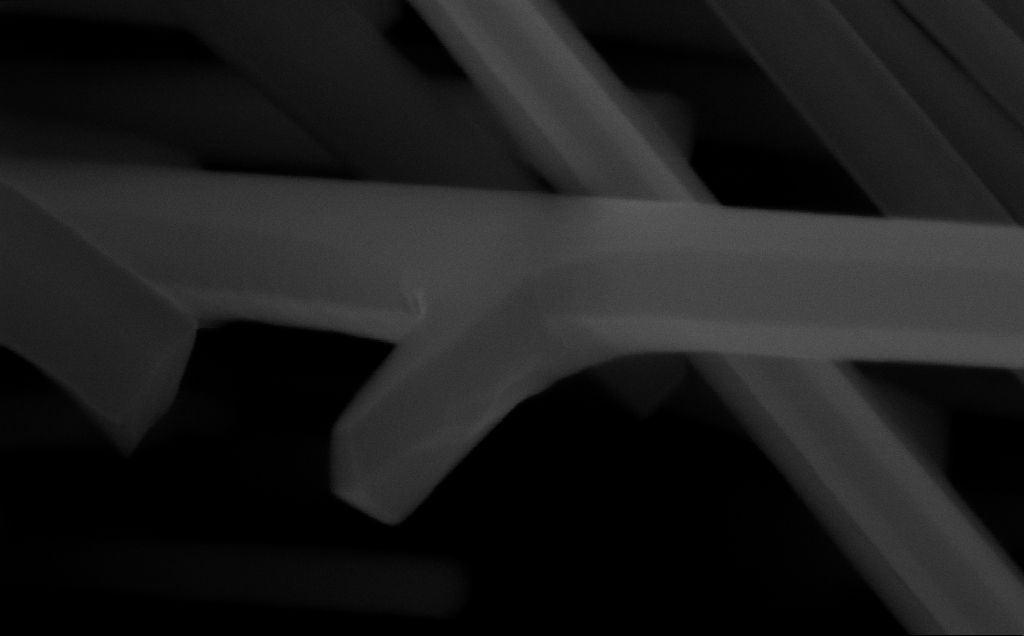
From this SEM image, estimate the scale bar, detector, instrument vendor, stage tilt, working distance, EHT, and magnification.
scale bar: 200 nm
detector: InLens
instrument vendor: Zeiss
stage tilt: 0°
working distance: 6 mm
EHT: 10 kV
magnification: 268.45 K X